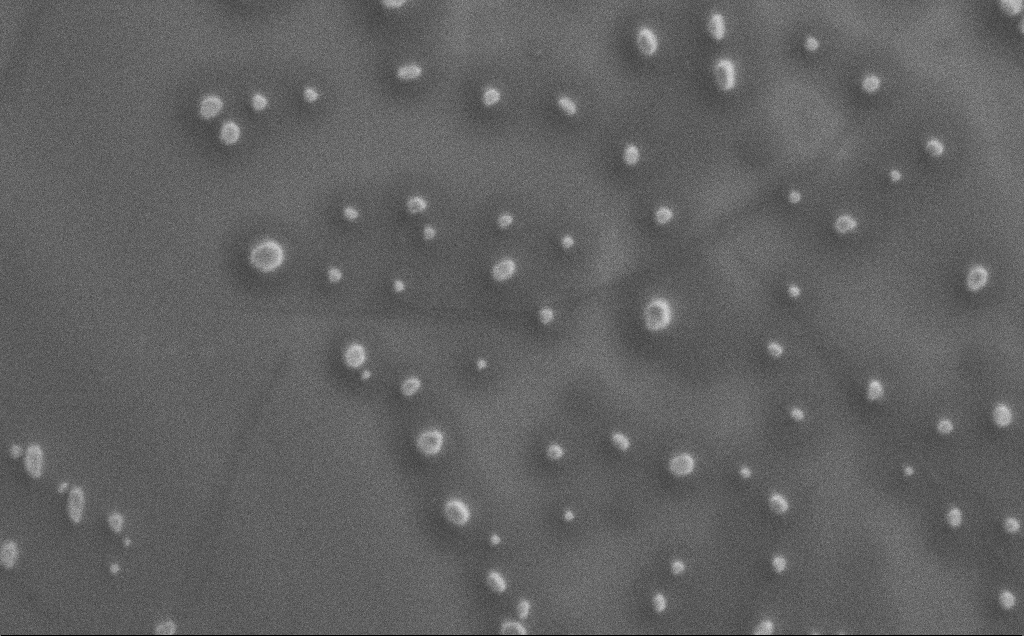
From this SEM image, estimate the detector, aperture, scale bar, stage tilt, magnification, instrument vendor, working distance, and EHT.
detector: SE2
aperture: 30 µm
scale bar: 2000 nm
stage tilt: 0°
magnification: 17.72 K X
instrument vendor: Zeiss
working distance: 3 mm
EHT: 1 kV